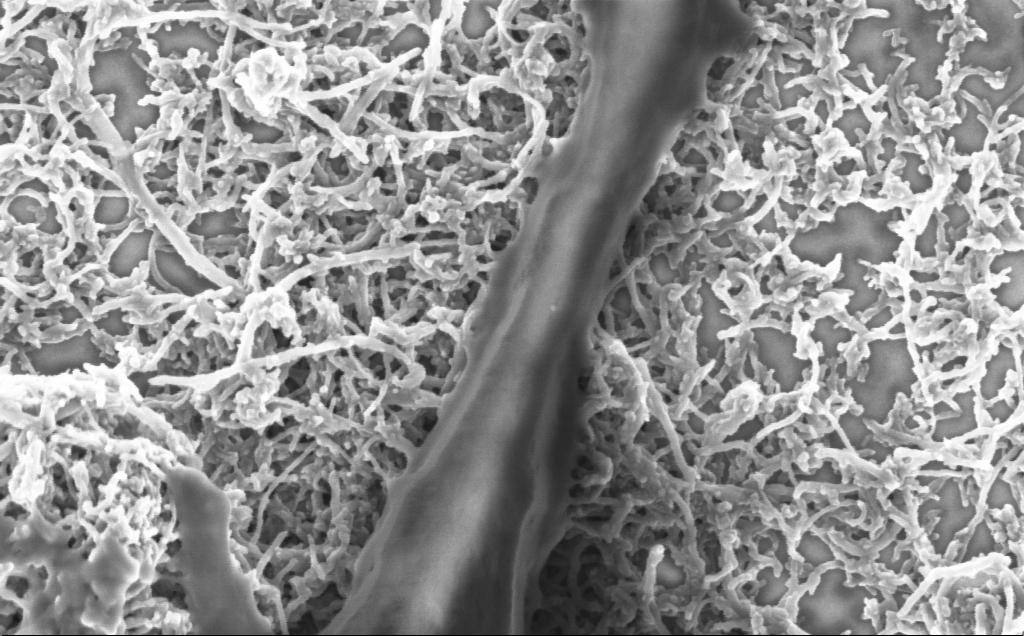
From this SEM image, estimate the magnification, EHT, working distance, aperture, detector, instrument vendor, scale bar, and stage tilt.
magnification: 75 K X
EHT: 2 kV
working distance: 7.1 mm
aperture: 30 µm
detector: InLens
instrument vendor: Zeiss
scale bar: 200 nm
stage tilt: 0°